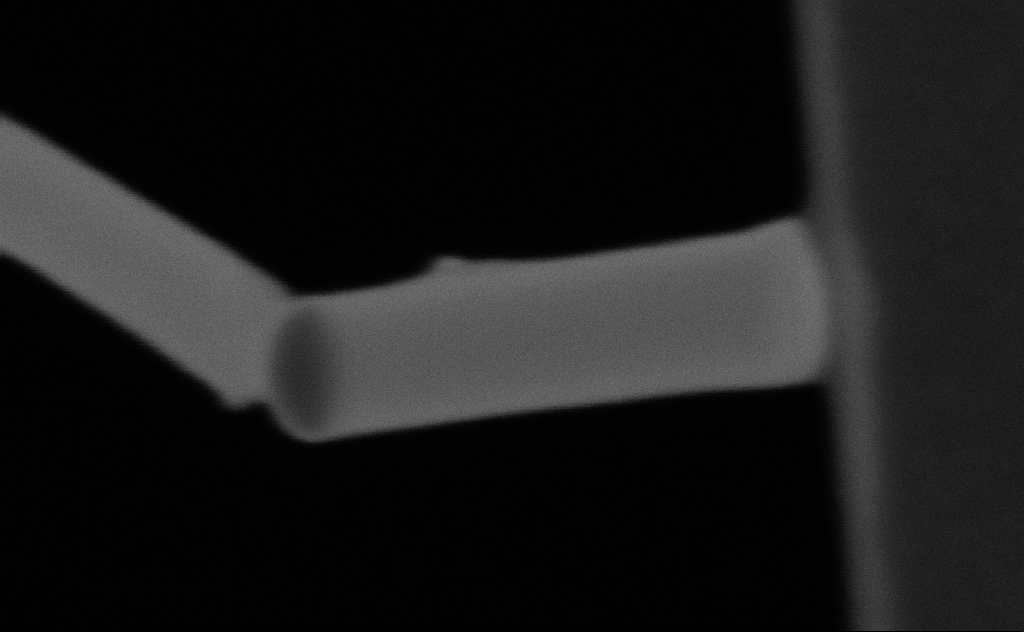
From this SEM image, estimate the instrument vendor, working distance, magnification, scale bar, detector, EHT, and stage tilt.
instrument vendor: Zeiss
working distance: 9 mm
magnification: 554.74 K X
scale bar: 100 nm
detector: SE2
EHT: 20 kV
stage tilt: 0°